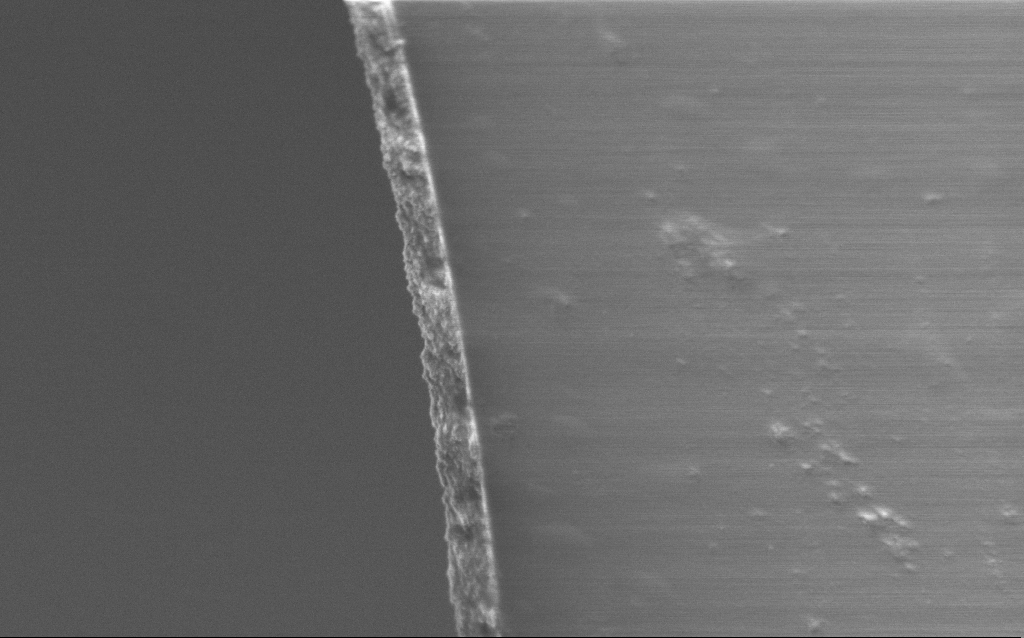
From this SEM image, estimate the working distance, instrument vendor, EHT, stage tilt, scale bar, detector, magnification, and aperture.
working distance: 5 mm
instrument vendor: Zeiss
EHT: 1 kV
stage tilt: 45°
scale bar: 1000 nm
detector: InLens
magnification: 45.89 K X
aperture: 30 µm